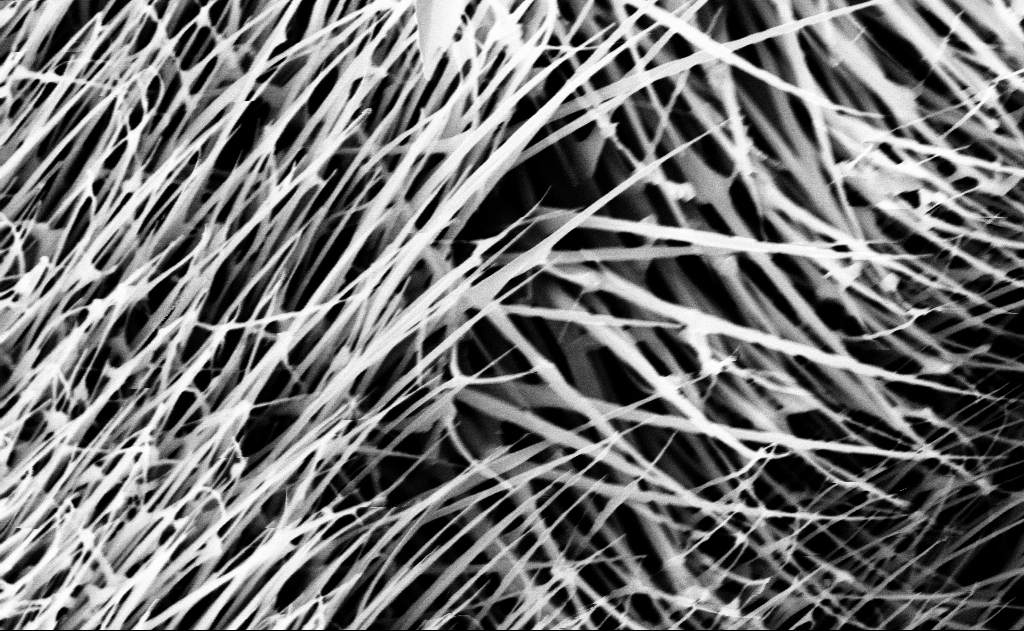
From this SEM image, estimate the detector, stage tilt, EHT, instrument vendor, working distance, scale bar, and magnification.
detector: InLens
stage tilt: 0°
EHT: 10 kV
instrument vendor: Zeiss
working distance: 13 mm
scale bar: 1000 nm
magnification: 40 K X